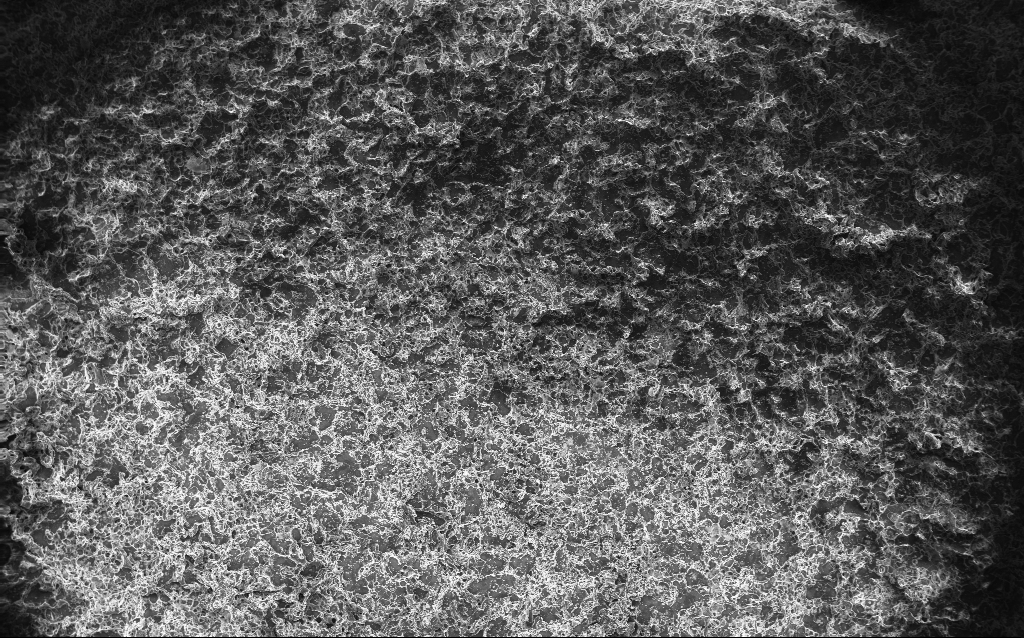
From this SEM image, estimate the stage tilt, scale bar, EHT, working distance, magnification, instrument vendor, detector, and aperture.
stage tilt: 0°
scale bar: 100000 nm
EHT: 10 kV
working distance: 2.7 mm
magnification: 0.122 K X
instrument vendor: Zeiss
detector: InLens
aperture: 30 µm